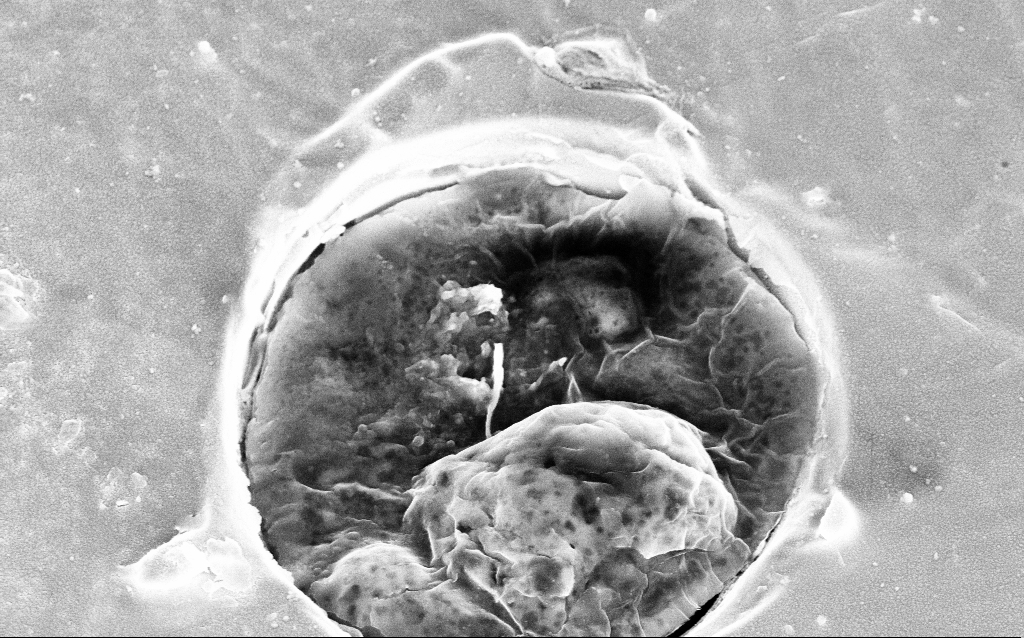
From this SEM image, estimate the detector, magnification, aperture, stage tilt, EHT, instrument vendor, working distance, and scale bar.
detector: InLens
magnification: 40.96 K X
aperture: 30 µm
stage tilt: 25.7°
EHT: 5 kV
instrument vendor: Zeiss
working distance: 6.9 mm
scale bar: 1000 nm